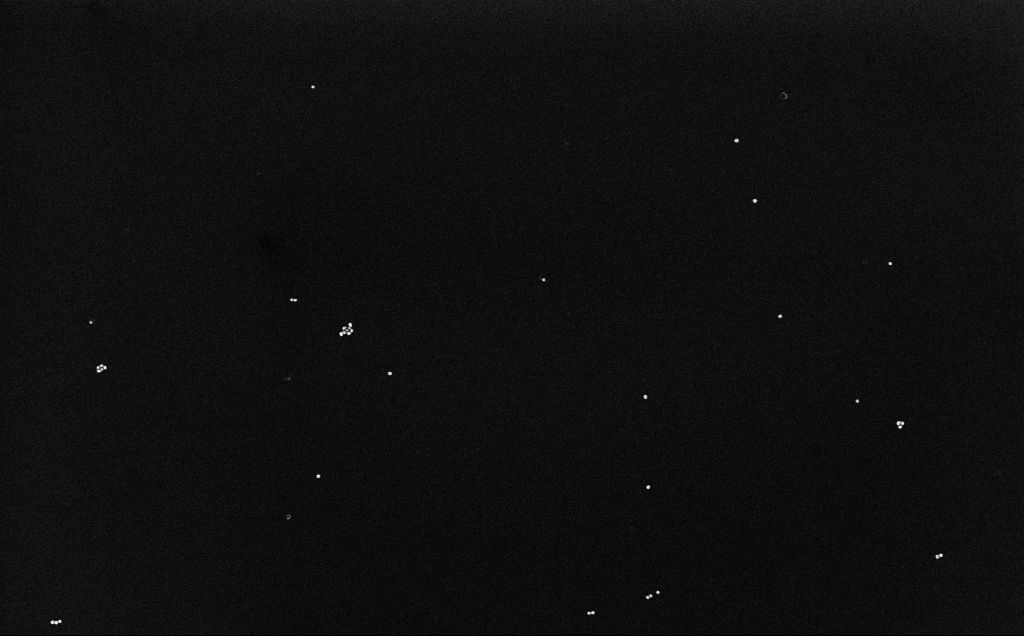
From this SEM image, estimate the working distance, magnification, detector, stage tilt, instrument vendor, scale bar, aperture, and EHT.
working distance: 6.6 mm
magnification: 100 K X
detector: InLens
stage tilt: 0°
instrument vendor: Zeiss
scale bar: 200 nm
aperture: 30 µm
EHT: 10 kV